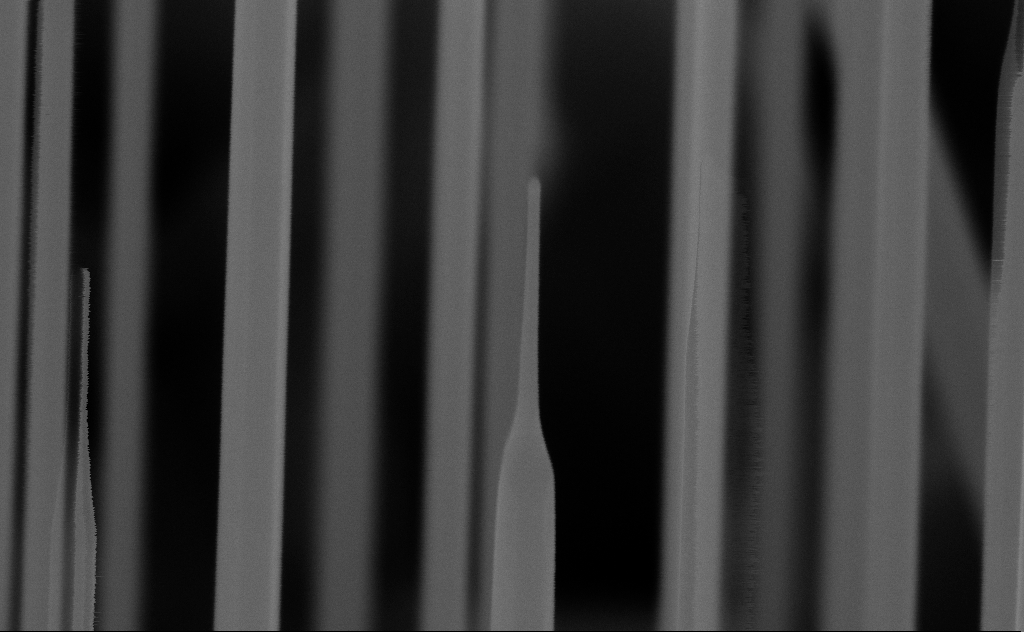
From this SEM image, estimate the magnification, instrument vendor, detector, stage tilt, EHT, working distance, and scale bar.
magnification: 220.85 K X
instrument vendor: Zeiss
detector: InLens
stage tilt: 44.9°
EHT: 10 kV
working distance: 7 mm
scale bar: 200 nm